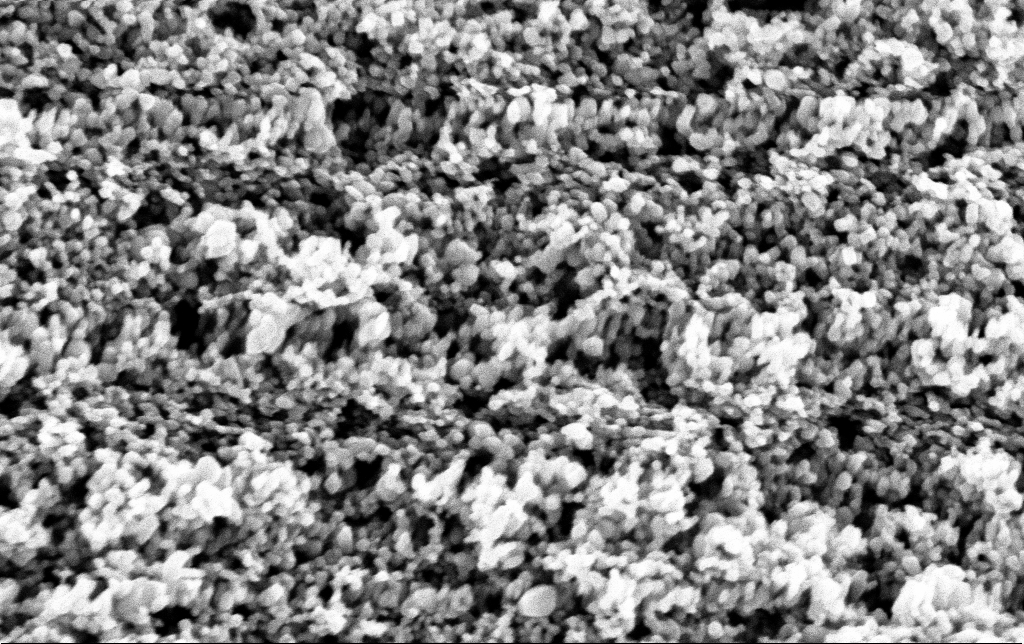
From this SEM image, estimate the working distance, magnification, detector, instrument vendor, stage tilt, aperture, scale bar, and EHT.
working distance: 2.8 mm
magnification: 155.62 K X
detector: InLens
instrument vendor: Zeiss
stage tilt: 0°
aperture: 30 µm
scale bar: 200 nm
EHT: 3 kV